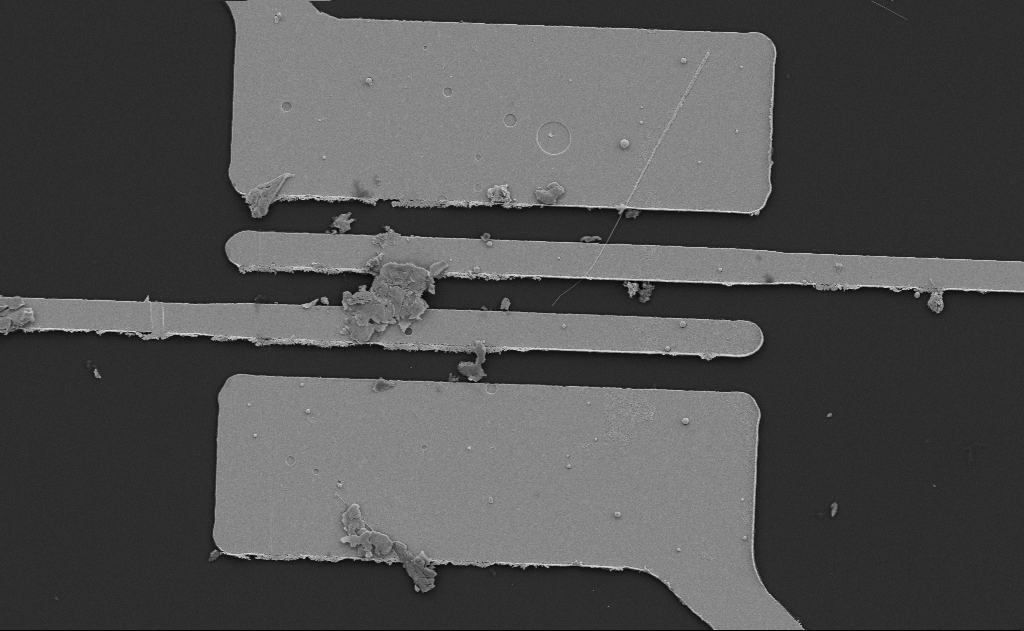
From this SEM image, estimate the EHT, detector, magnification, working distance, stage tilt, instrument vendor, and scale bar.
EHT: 5 kV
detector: SE2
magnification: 6.65 K X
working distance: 13 mm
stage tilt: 0°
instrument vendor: Zeiss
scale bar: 10000 nm